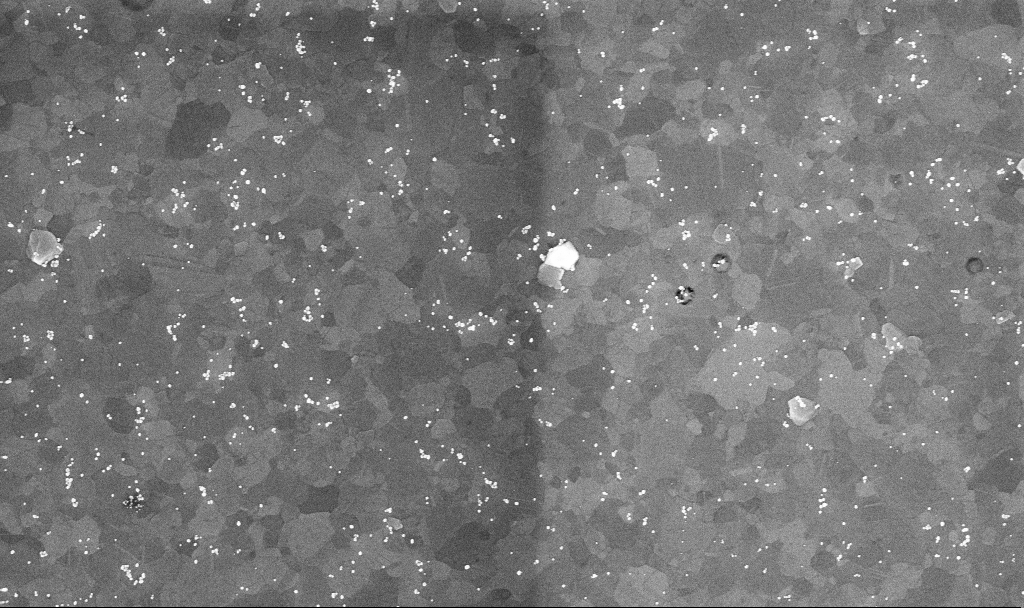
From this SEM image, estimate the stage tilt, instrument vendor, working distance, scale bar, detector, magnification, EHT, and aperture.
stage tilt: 0°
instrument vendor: Zeiss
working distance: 3.4 mm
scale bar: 1000 nm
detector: InLens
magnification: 50.42 K X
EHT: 10 kV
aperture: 30 µm